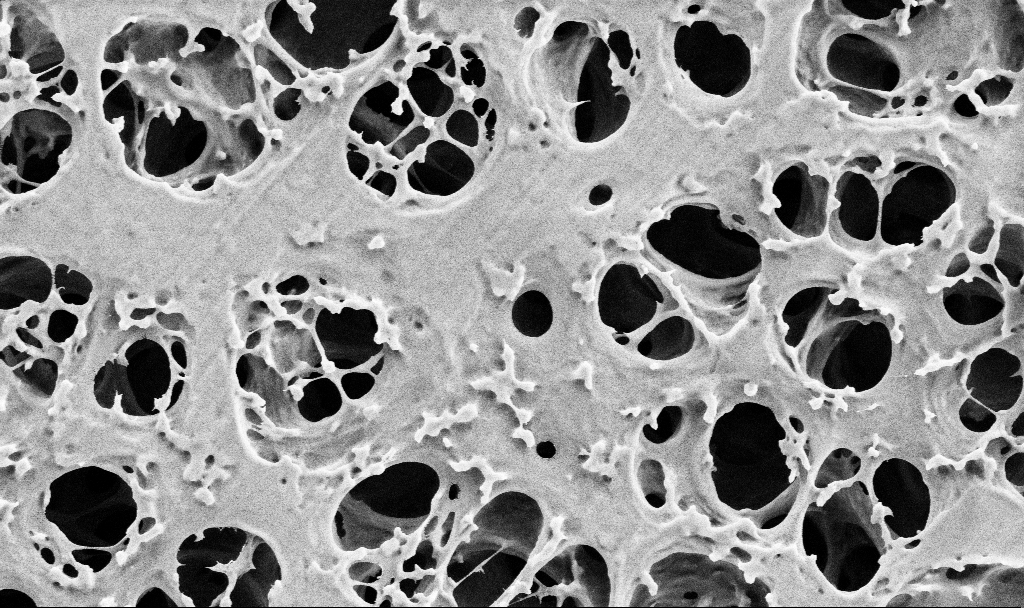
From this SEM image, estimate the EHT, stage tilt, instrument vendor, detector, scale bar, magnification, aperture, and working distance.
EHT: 2 kV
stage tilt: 0°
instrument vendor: Zeiss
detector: SE2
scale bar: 2000 nm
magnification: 25 K X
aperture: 30 µm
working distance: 3.7 mm